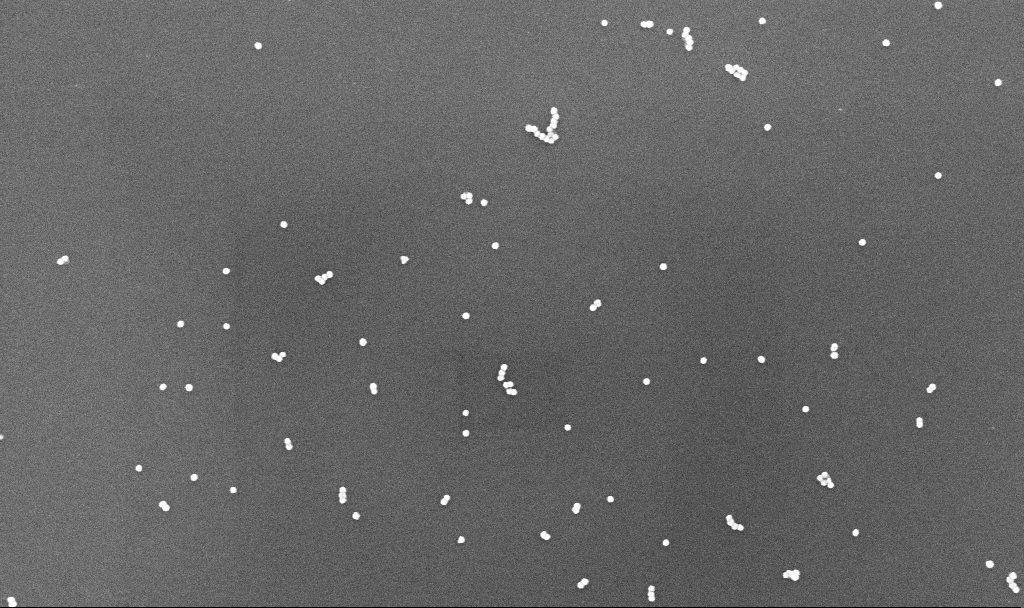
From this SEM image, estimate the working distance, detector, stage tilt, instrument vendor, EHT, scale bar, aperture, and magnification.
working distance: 3.4 mm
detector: InLens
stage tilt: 0°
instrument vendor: Zeiss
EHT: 10 kV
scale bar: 200 nm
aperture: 30 µm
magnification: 100 K X